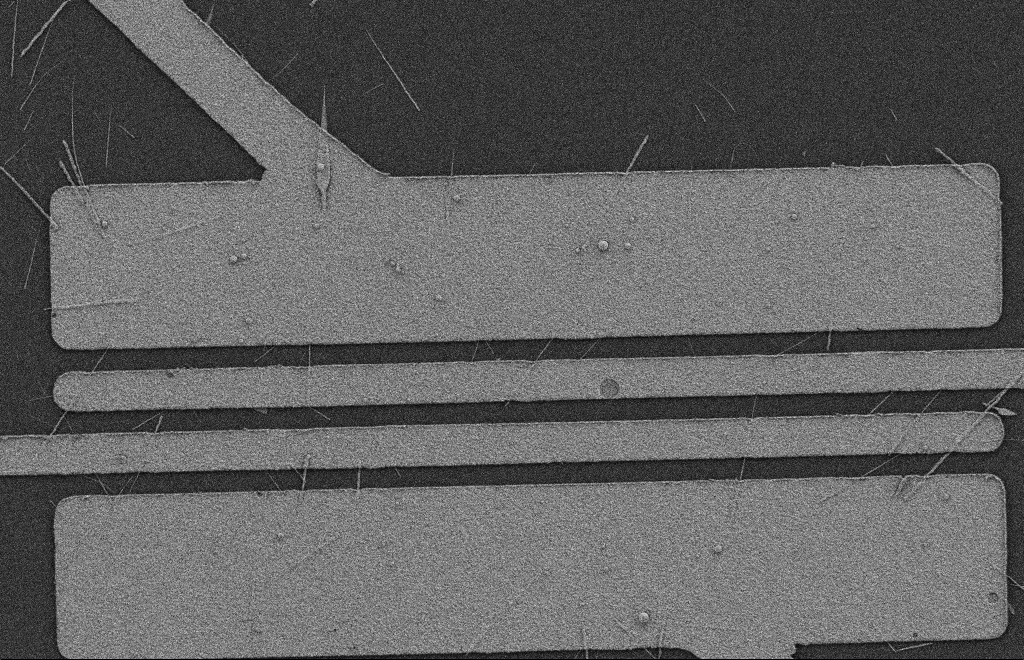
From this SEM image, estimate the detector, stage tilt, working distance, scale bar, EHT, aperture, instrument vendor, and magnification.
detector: SE2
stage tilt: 0°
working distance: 9 mm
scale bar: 2000 nm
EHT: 2 kV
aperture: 20 µm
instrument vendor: Zeiss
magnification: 5.71 K X